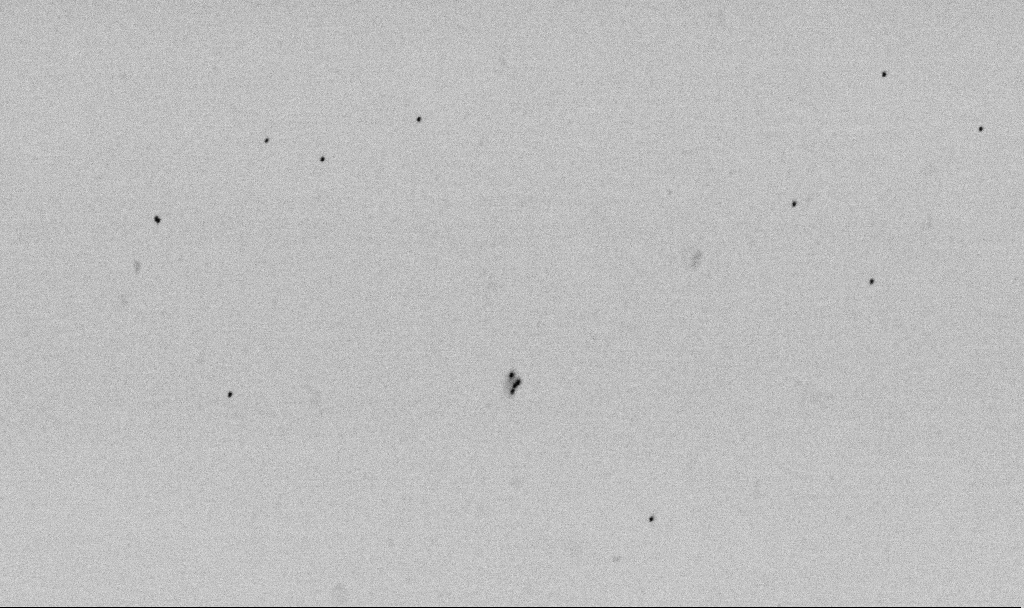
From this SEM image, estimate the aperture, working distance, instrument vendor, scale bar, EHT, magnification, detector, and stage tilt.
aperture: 30 µm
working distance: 6.5 mm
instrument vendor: Zeiss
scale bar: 1000 nm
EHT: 2 kV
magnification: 50 K X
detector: SE2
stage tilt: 0°